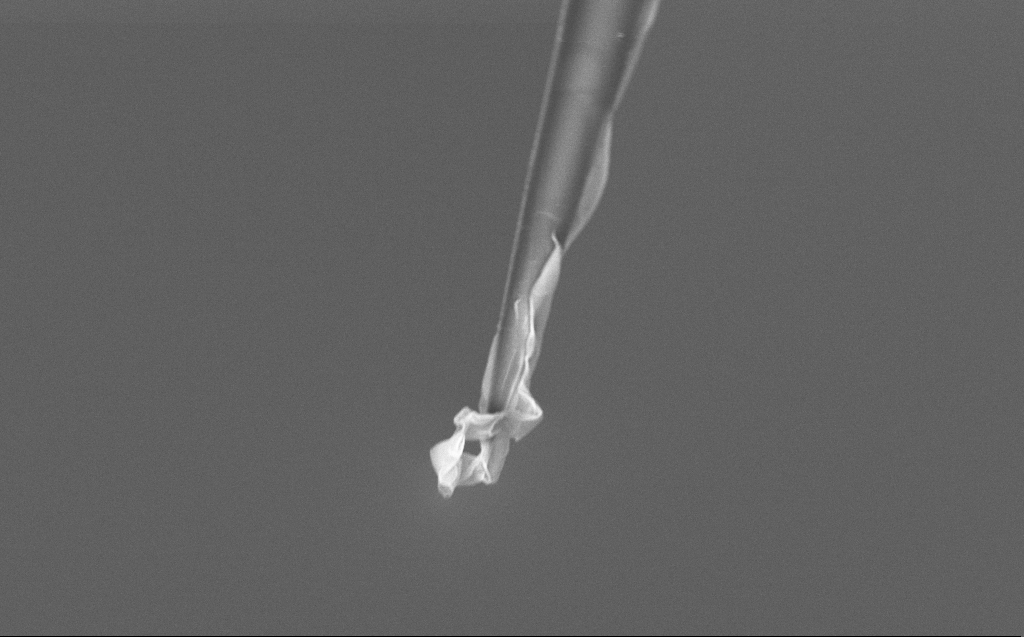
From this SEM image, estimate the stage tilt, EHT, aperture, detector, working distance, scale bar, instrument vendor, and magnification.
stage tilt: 45°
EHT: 5 kV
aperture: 30 µm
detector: InLens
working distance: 6 mm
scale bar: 2000 nm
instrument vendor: Zeiss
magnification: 10 K X